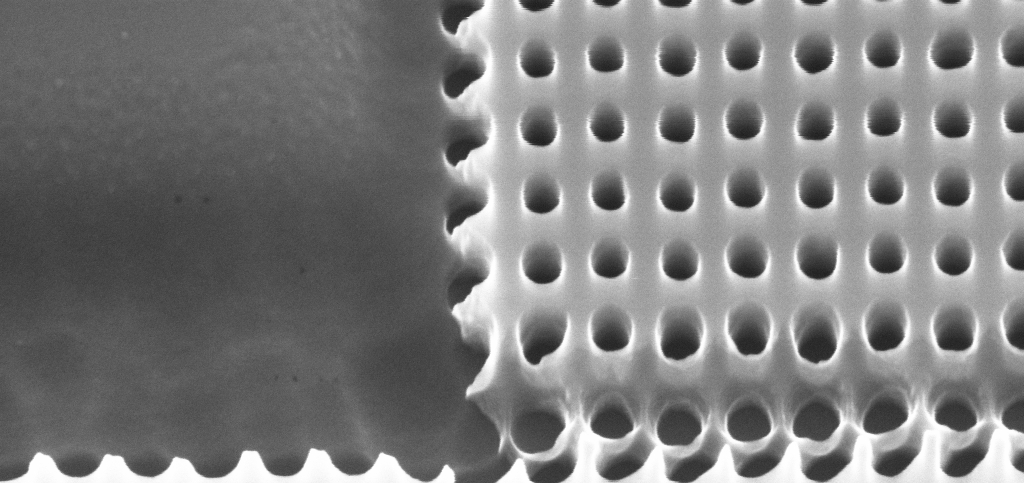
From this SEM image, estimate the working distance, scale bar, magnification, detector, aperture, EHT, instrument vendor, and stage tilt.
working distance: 4 mm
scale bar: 200 nm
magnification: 168.54 K X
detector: InLens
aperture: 30 µm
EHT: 10 kV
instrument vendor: Zeiss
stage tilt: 30°